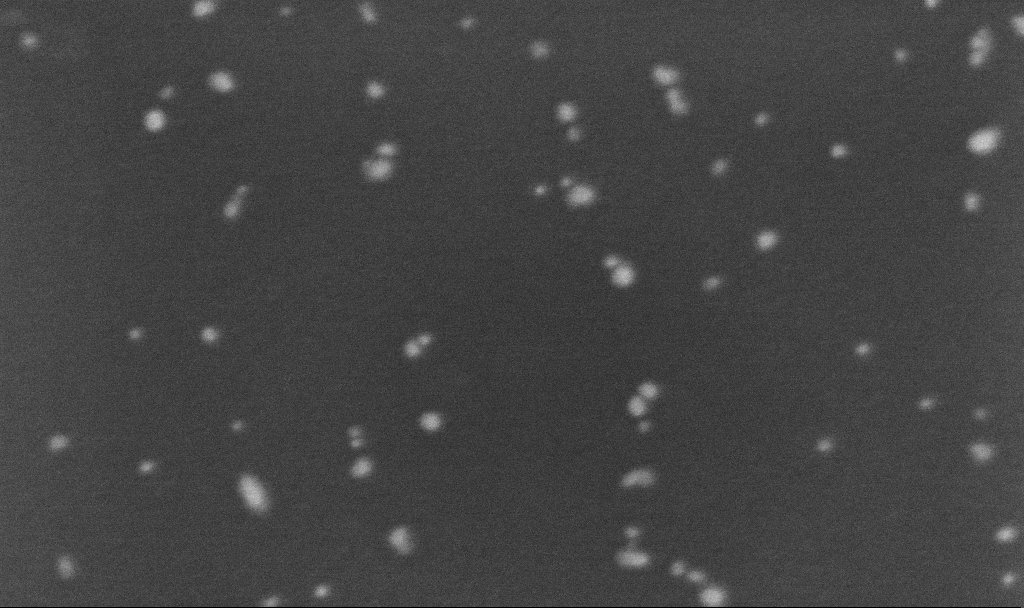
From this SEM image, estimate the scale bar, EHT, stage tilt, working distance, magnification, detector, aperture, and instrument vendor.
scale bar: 100 nm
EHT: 5 kV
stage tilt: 0°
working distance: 3.2 mm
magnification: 400 K X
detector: InLens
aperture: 30 µm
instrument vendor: Zeiss